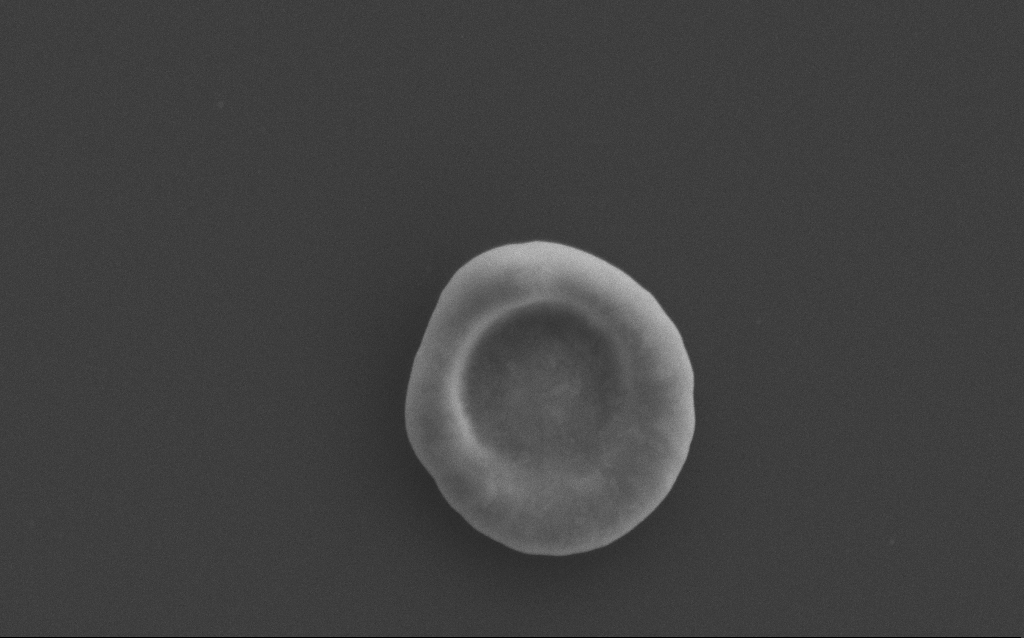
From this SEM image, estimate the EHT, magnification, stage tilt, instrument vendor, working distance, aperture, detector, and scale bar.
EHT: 5 kV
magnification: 49.99 K X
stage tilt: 0°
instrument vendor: Zeiss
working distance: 4 mm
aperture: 30 µm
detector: SE2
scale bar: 1000 nm